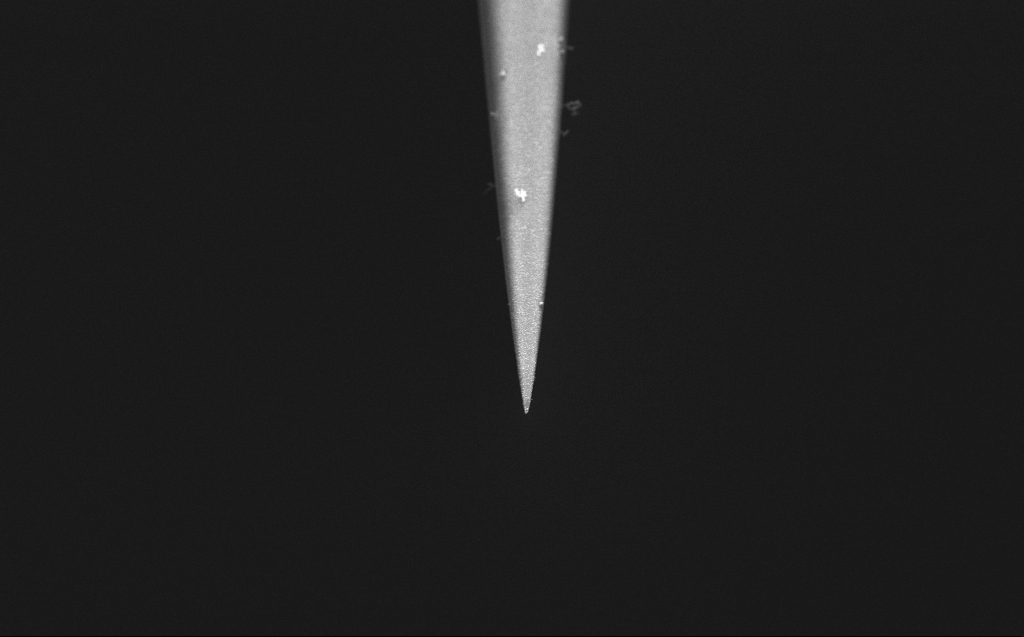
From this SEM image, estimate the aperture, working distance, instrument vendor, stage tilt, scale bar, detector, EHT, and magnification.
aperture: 30 µm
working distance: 3 mm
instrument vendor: Zeiss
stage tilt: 45°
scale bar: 2000 nm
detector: InLens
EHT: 5 kV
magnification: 10 K X